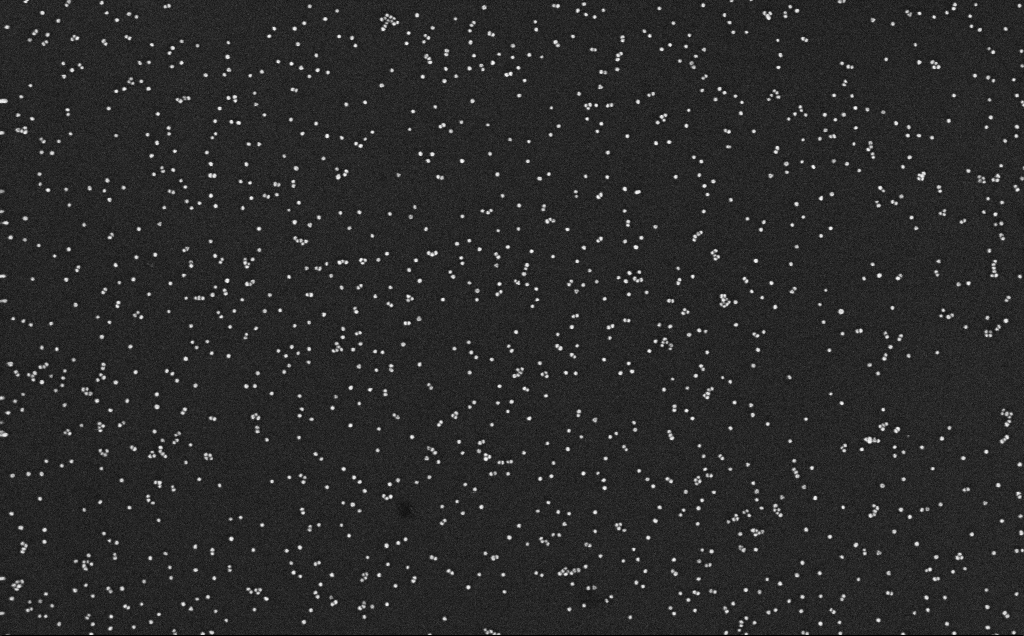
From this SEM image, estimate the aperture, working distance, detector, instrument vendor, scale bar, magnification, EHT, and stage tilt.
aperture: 30 µm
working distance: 3.1 mm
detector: InLens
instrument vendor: Zeiss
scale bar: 200 nm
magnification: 100 K X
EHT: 10 kV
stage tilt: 0°